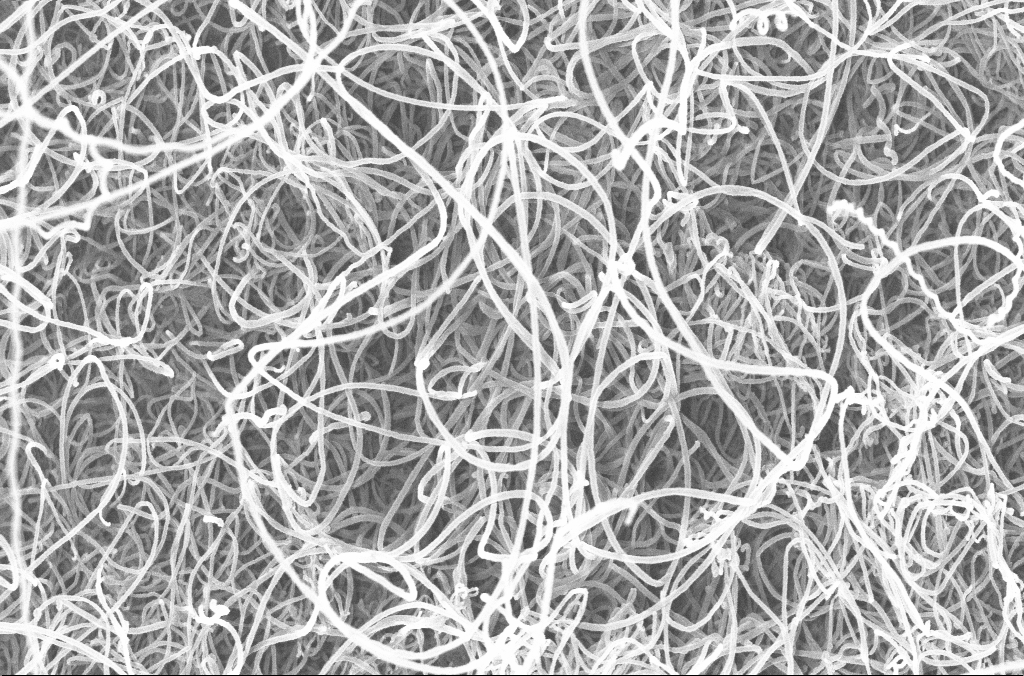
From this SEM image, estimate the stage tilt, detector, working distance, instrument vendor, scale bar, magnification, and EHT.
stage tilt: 0°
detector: InLens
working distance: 4.2 mm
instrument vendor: Zeiss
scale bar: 1000 nm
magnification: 50 K X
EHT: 20 kV